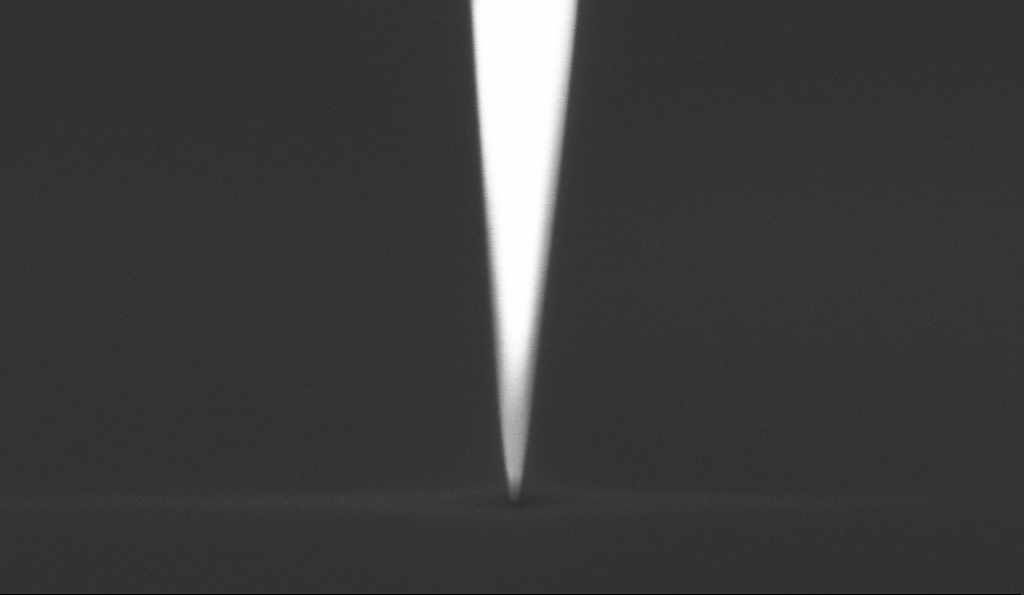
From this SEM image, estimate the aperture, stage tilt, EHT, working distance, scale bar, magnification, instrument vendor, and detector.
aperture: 30 µm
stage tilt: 0°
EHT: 1 kV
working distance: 5.4 mm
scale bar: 200 nm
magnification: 100 K X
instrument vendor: Zeiss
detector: InLens